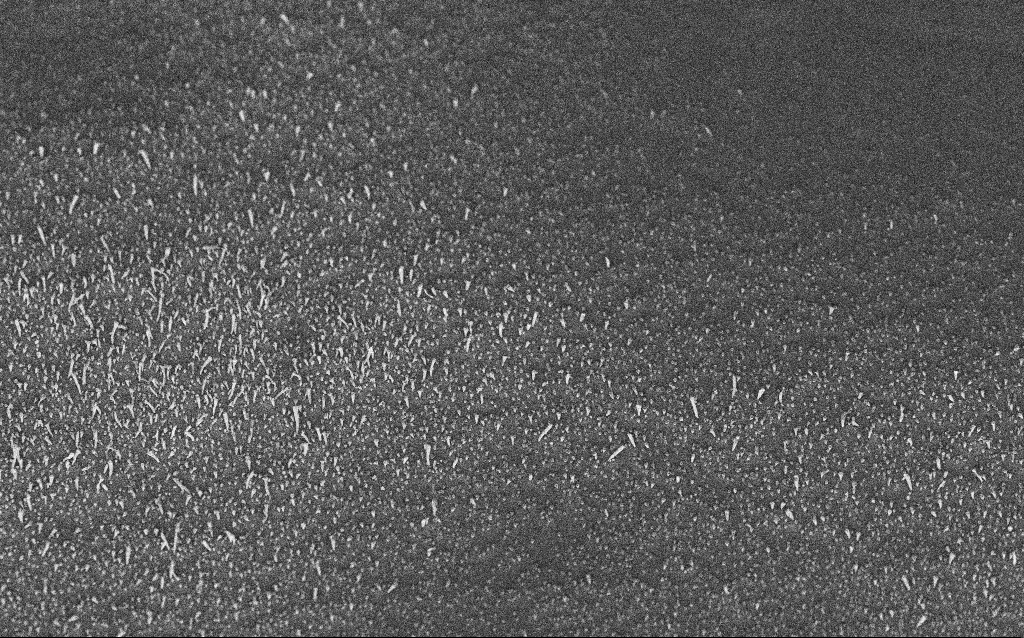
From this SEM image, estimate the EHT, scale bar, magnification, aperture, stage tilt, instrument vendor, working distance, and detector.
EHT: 5 kV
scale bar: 2000 nm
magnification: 10 K X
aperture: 30 µm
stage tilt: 45°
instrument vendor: Zeiss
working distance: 6.7 mm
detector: InLens